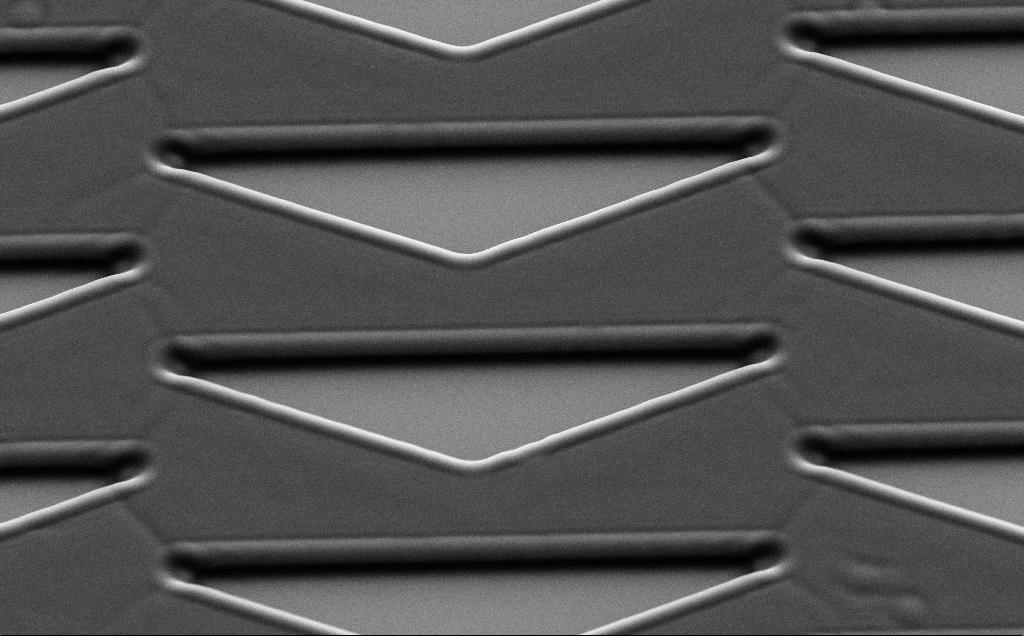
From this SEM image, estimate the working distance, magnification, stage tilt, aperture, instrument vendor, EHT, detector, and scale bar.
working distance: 6 mm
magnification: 5.52 K X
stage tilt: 35°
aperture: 30 µm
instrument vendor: Zeiss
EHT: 7 kV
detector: SE2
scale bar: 10000 nm